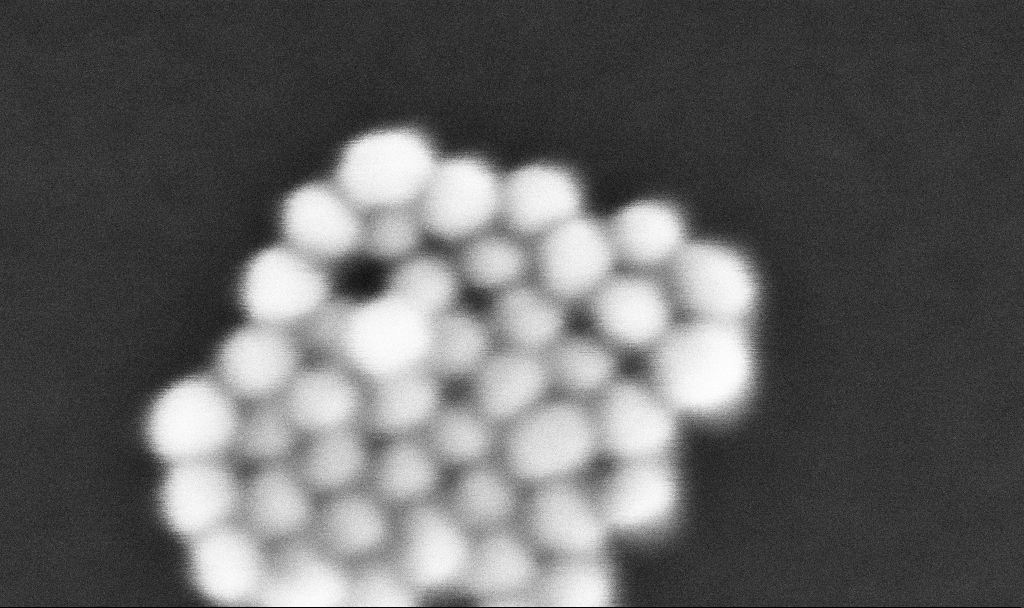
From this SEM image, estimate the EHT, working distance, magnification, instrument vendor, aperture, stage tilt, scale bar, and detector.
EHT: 10 kV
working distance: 3.3 mm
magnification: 1379.28 K X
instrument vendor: Zeiss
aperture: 30 µm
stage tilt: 0°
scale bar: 20 nm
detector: InLens